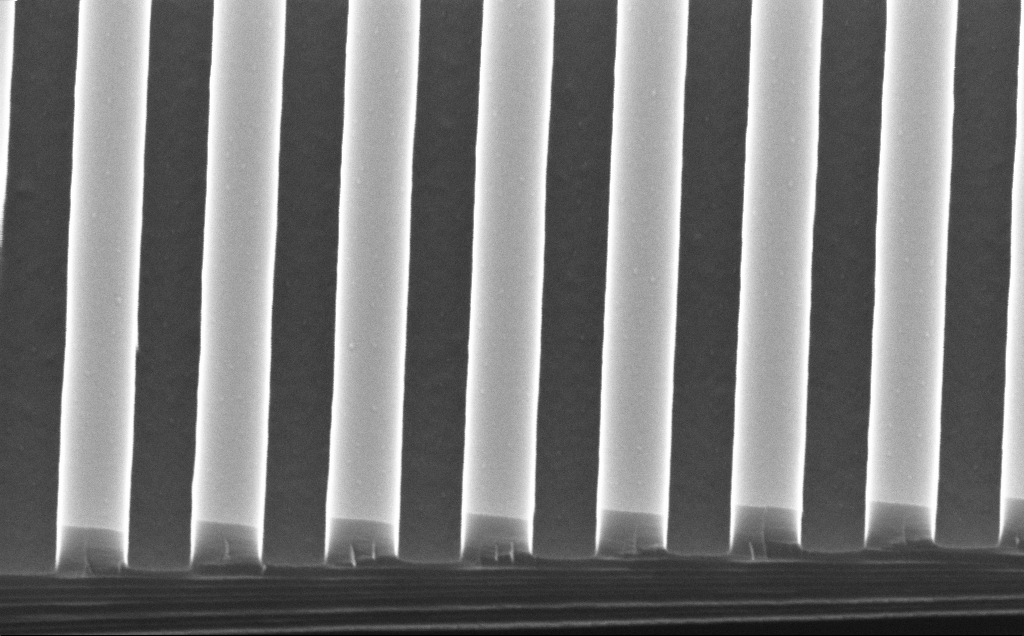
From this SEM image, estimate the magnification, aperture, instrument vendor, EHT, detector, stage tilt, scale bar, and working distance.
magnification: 100 K X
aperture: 30 µm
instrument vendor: Zeiss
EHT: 10 kV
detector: InLens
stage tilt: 45°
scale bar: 200 nm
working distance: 4 mm